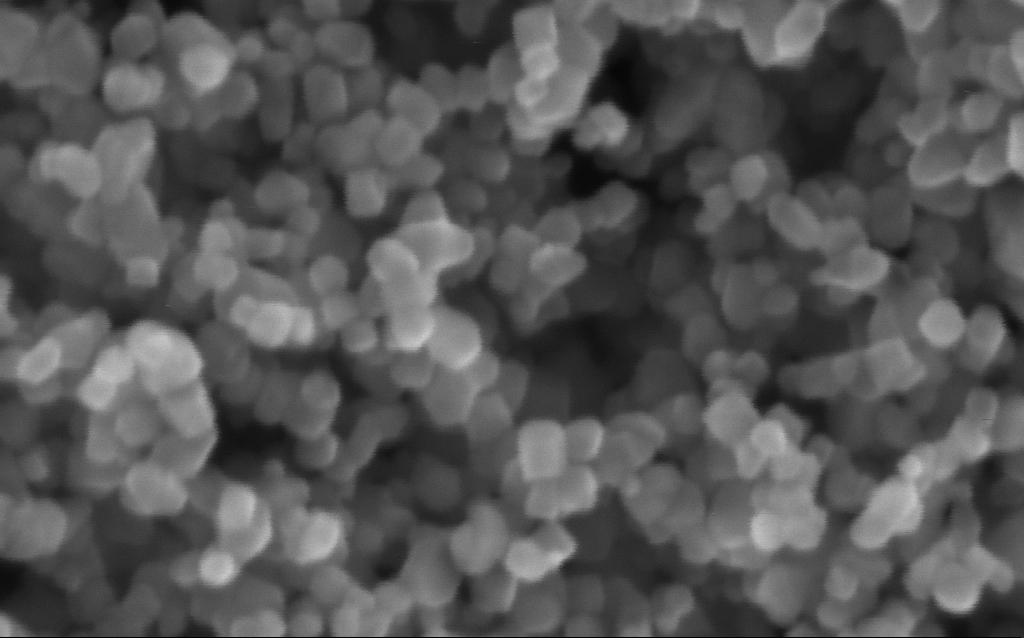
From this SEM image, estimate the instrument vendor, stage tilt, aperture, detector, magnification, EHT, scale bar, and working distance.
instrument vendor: Zeiss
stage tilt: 0°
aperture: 30 µm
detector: InLens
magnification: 716 K X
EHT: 5 kV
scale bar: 100 nm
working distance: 3 mm